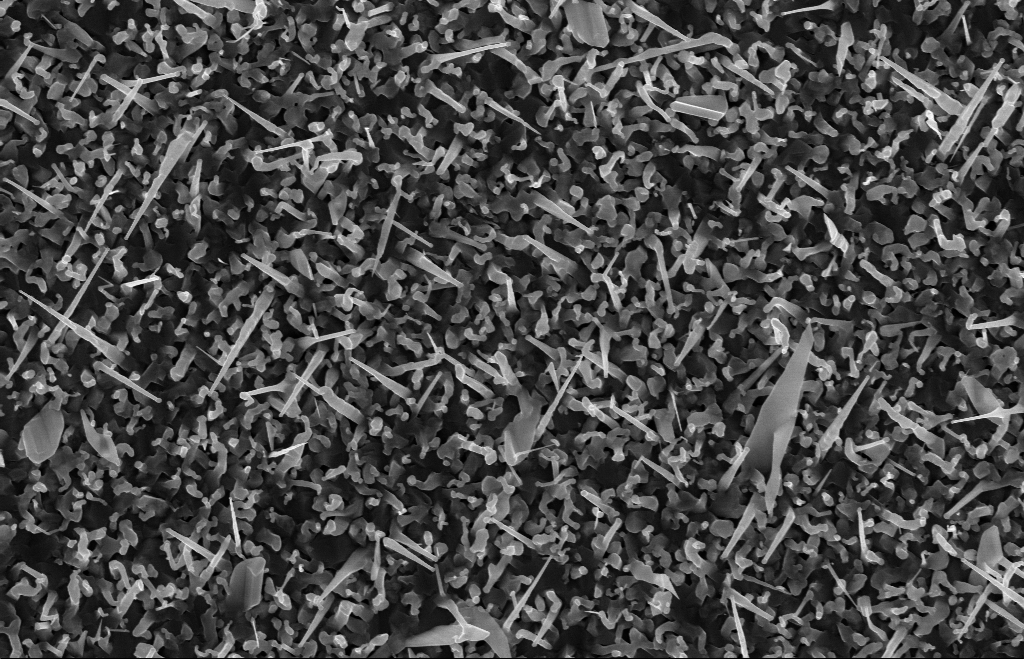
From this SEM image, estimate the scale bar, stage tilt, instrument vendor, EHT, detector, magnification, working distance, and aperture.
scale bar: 1000 nm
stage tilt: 0°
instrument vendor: Zeiss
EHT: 10 kV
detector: InLens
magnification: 20 K X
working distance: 8 mm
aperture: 30 µm